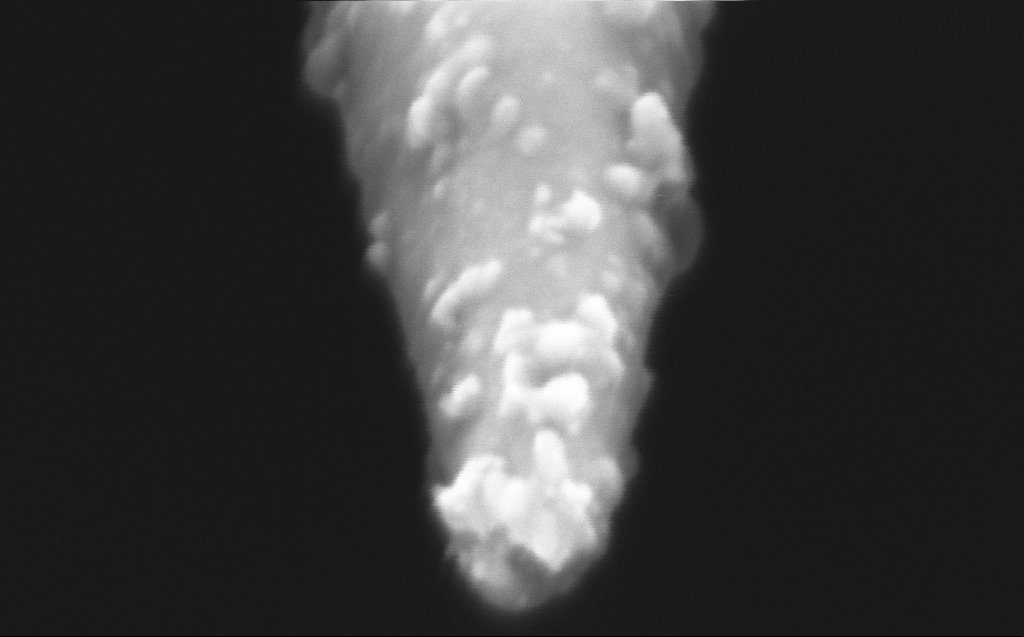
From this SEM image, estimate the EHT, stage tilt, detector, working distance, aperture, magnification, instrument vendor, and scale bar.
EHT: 2 kV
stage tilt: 45°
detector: InLens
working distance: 4 mm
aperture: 30 µm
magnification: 500 K X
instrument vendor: Zeiss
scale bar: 100 nm